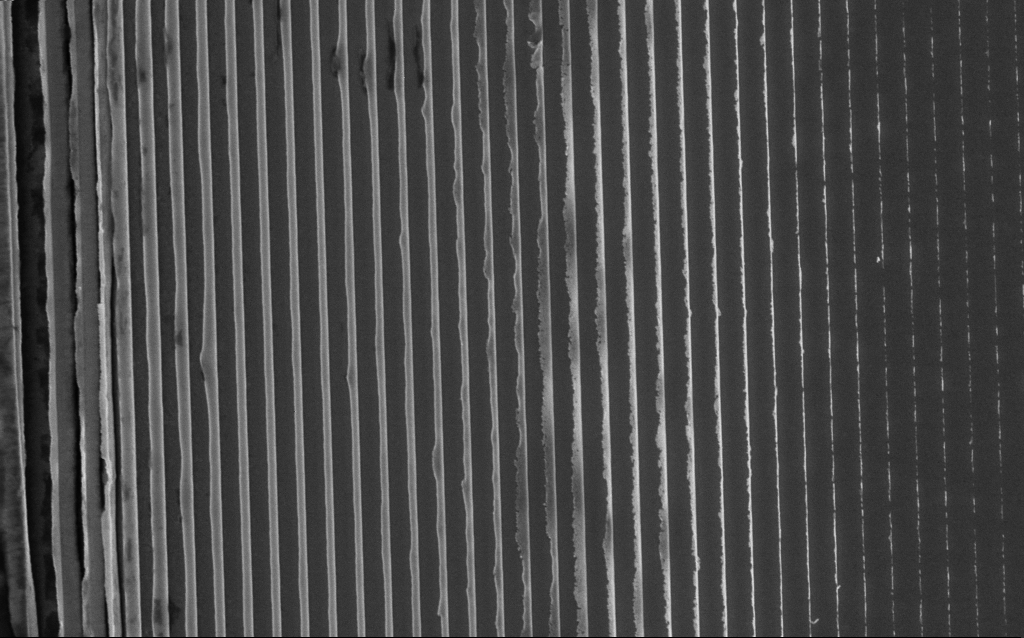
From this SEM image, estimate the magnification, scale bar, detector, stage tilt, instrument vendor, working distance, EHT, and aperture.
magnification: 20.62 K X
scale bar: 2000 nm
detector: InLens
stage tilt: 0°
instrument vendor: Zeiss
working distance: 8 mm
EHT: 10 kV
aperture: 30 µm